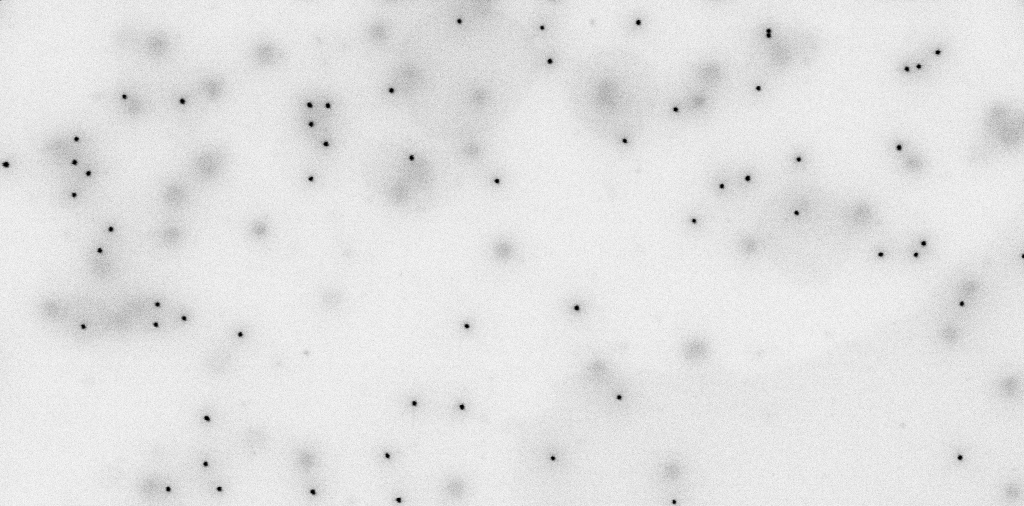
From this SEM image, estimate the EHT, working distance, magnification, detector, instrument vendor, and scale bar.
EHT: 2 kV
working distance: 4.9 mm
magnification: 69.64 K X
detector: SE2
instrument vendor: Zeiss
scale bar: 200 nm